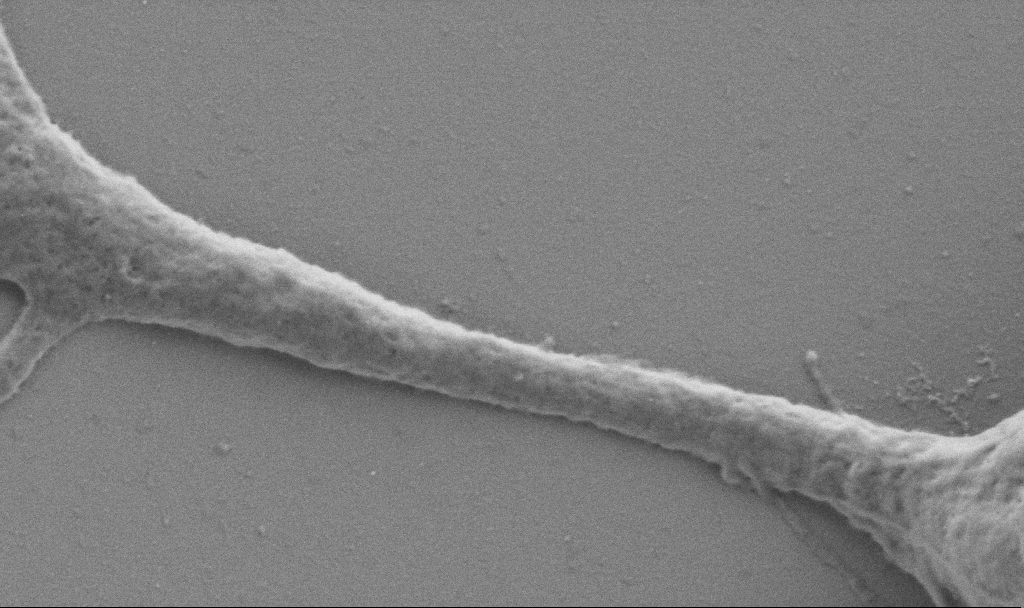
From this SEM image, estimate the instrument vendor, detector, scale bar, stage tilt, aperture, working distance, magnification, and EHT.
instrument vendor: Zeiss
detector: SE2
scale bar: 1000 nm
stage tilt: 0°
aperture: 30 µm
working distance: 6.9 mm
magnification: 50 K X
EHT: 0.9 kV